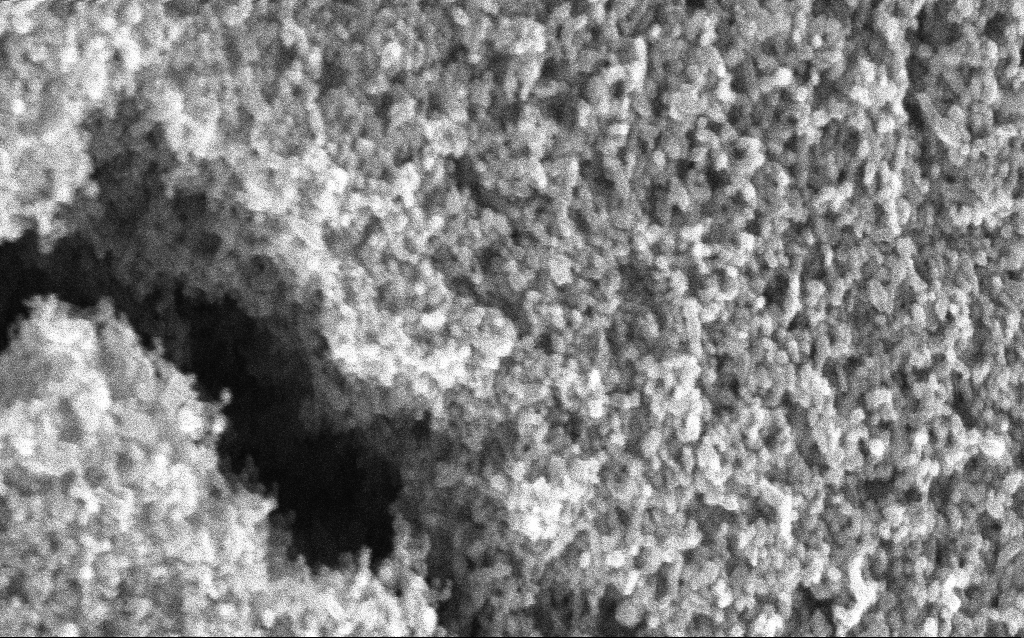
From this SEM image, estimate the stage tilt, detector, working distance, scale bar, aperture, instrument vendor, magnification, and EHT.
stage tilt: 0°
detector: InLens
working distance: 2.6 mm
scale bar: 100 nm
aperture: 30 µm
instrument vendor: Zeiss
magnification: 211.33 K X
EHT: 5 kV